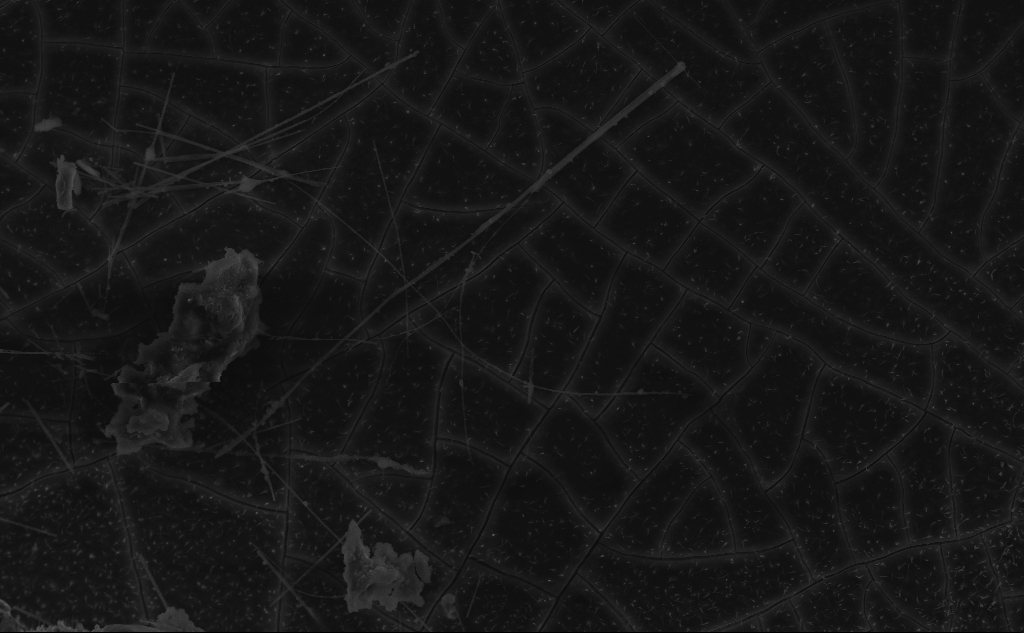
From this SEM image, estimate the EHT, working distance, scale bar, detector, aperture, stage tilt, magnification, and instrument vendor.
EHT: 10 kV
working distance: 6 mm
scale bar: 10000 nm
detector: InLens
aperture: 30 µm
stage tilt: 45°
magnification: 3.34 K X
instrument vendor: Zeiss